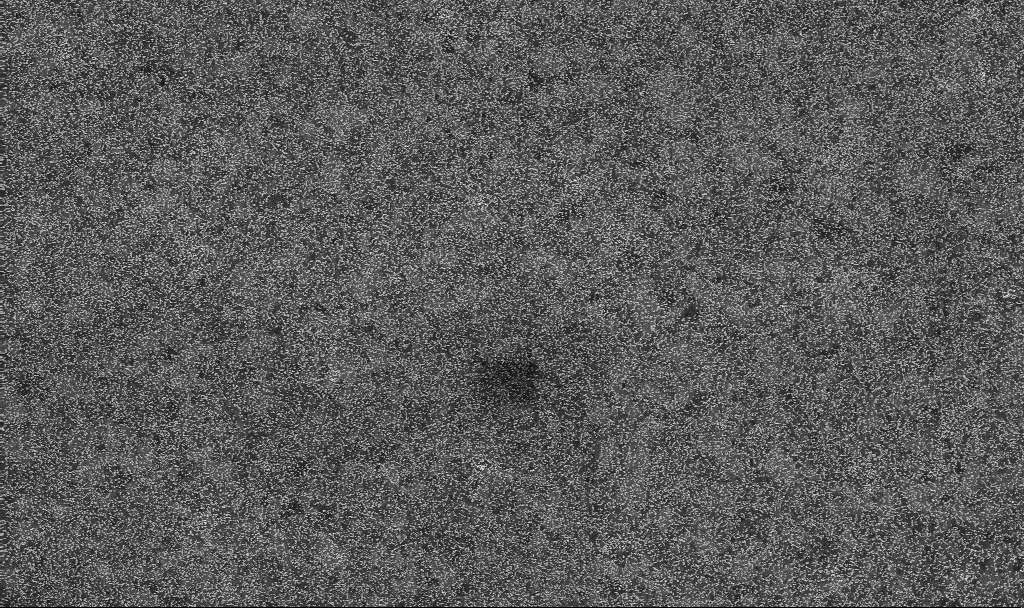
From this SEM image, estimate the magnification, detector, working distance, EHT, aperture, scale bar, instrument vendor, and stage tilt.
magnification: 20 K X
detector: InLens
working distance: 3.3 mm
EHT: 10 kV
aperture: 30 µm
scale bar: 1000 nm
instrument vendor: Zeiss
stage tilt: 0°